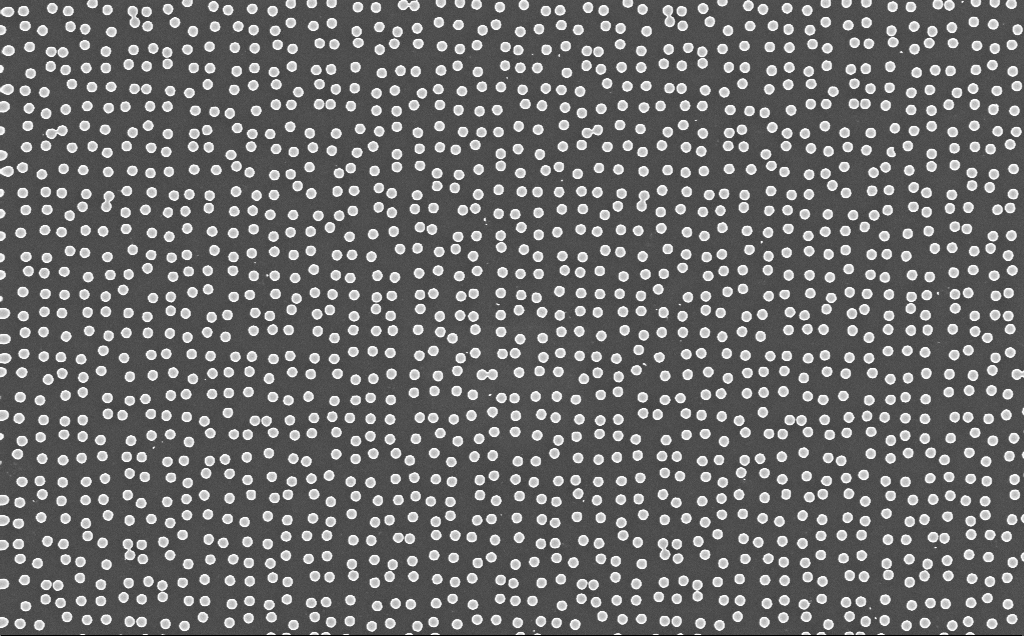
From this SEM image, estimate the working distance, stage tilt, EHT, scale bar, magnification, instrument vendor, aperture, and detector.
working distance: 6 mm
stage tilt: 0°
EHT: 10 kV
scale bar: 1000 nm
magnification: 18.94 K X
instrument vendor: Zeiss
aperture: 30 µm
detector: InLens